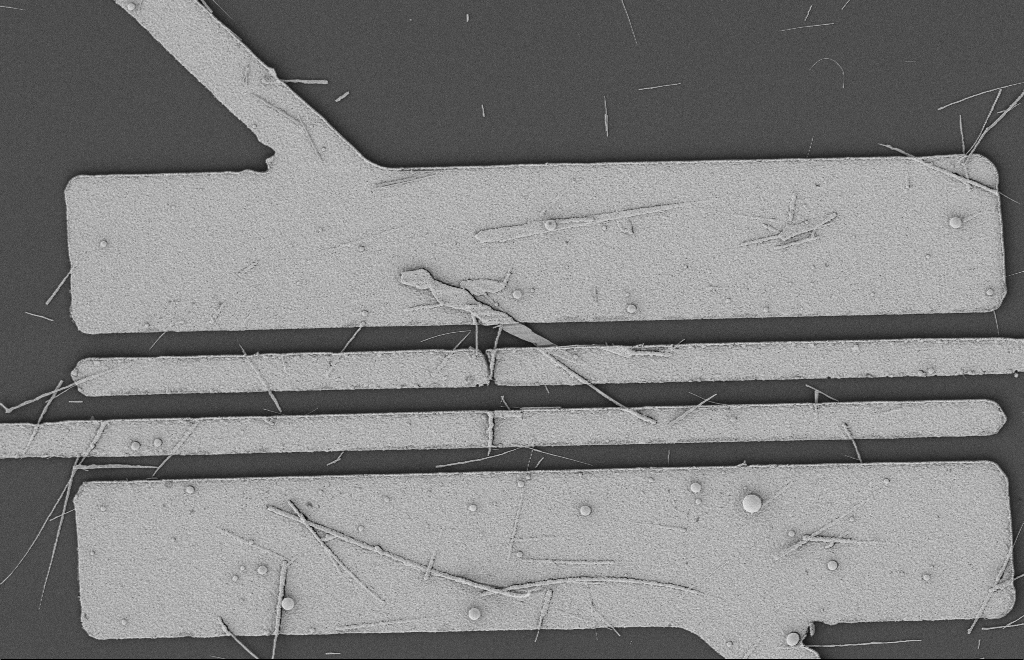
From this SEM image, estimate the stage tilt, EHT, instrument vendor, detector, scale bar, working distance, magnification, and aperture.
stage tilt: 0°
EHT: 2 kV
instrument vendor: Zeiss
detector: SE2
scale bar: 2000 nm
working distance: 12 mm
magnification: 5.59 K X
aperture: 20 µm